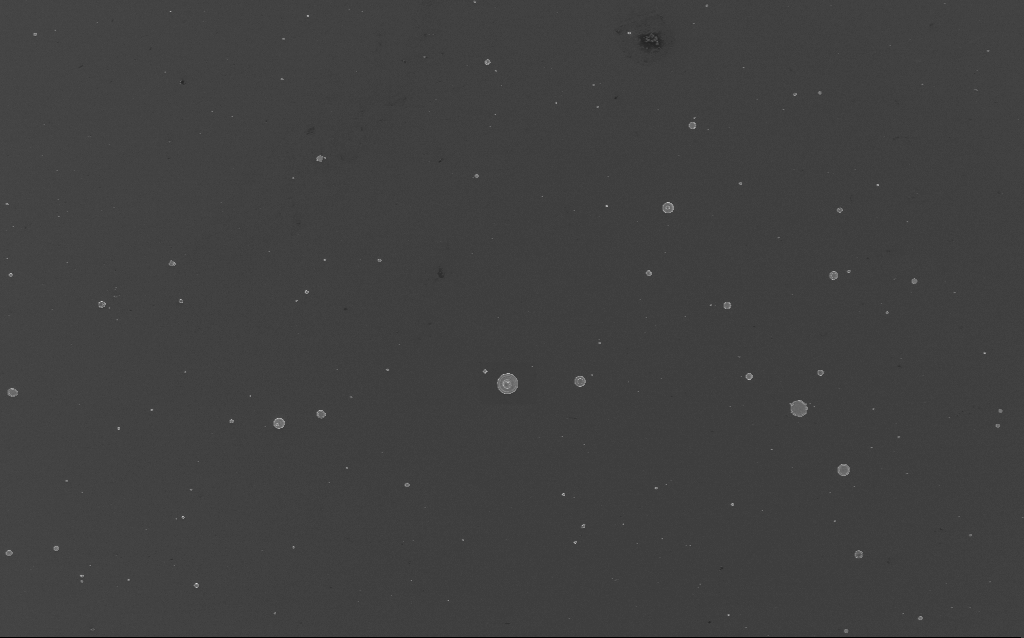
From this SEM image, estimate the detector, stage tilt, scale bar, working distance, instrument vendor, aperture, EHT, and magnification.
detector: InLens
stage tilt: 0°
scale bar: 10000 nm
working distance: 3 mm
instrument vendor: Zeiss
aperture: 30 µm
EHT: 3 kV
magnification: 1.65 K X